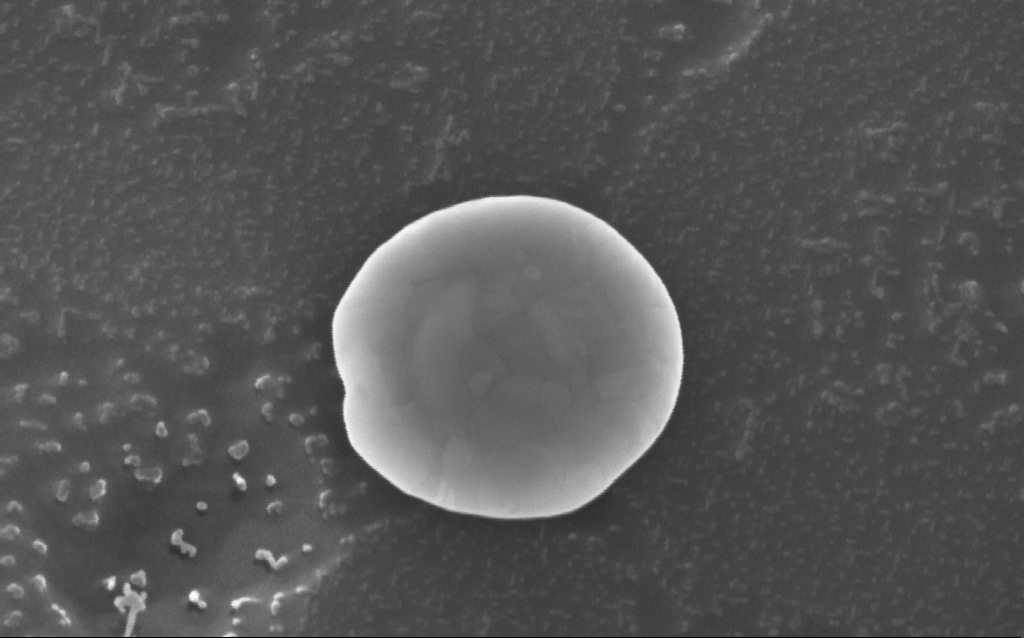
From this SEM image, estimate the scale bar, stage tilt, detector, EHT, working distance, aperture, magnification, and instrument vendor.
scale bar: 100 nm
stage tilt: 21.3°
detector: InLens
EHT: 10 kV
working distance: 6 mm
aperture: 30 µm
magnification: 213.56 K X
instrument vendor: Zeiss